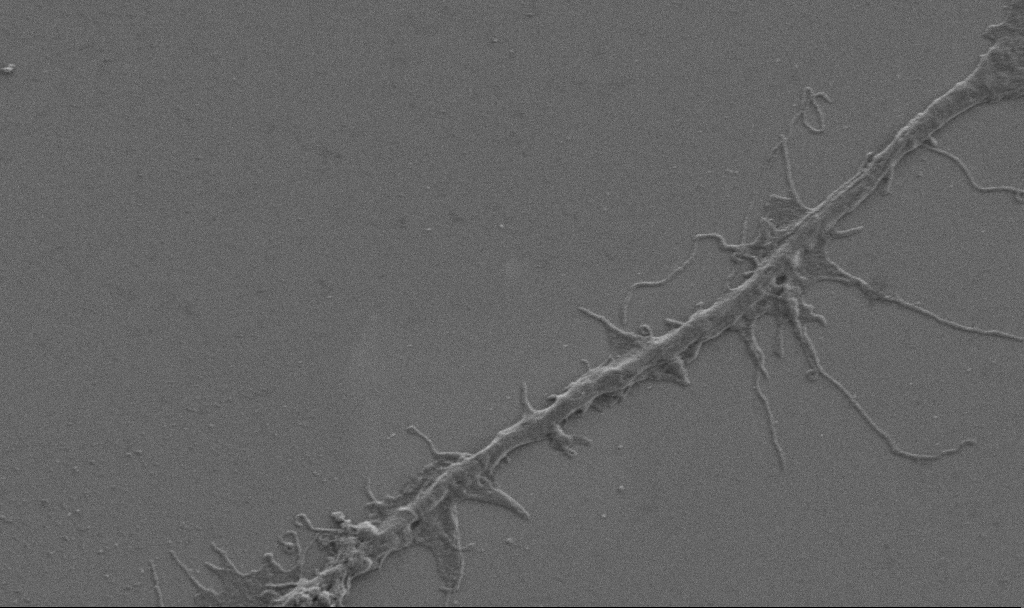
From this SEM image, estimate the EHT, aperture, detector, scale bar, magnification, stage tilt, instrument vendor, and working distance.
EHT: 1 kV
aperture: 30 µm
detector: SE2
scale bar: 2000 nm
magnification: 10 K X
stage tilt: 0°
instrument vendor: Zeiss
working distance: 6.9 mm